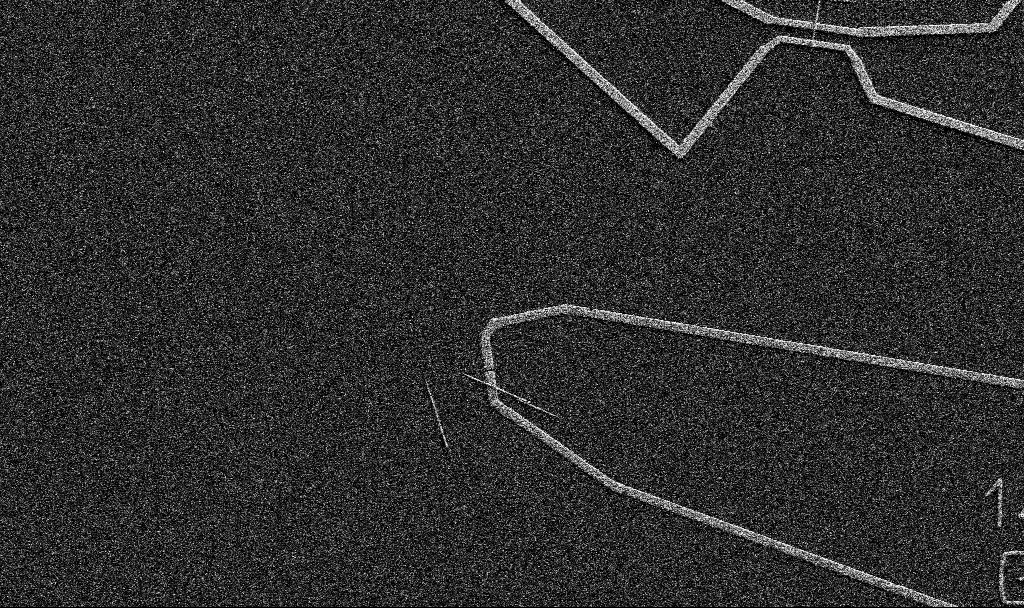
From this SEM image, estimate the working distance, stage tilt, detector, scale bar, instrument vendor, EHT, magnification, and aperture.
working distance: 10.7 mm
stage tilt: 0°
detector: SE2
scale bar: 10000 nm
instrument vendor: Zeiss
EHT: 5 kV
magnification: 5 K X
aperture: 30 µm